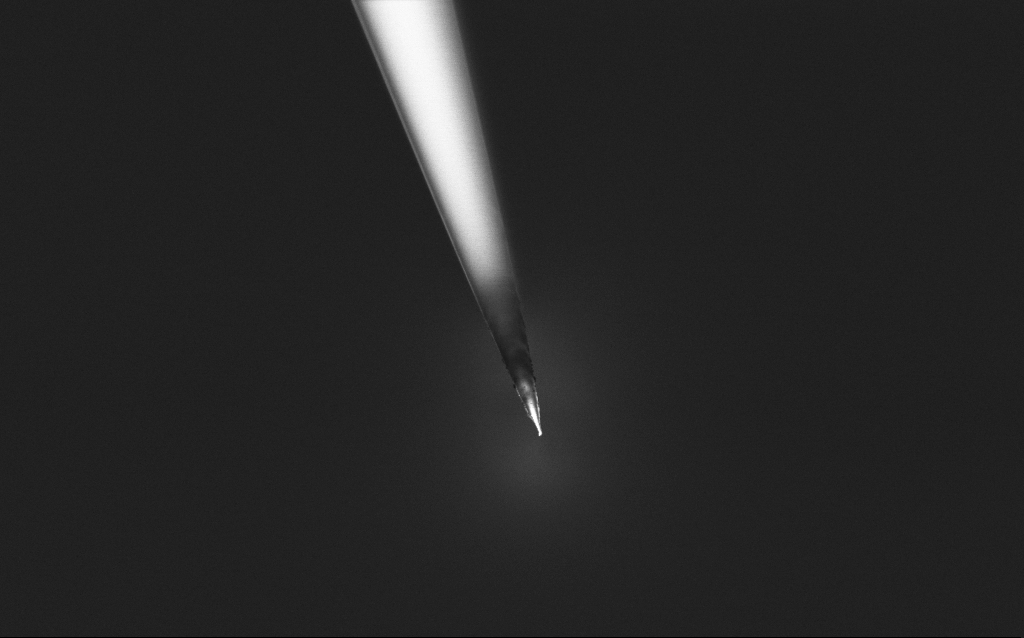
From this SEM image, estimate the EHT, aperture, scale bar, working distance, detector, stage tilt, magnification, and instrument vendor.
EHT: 1 kV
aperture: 30 µm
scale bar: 2000 nm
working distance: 6 mm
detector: InLens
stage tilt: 45°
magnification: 10 K X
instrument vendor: Zeiss